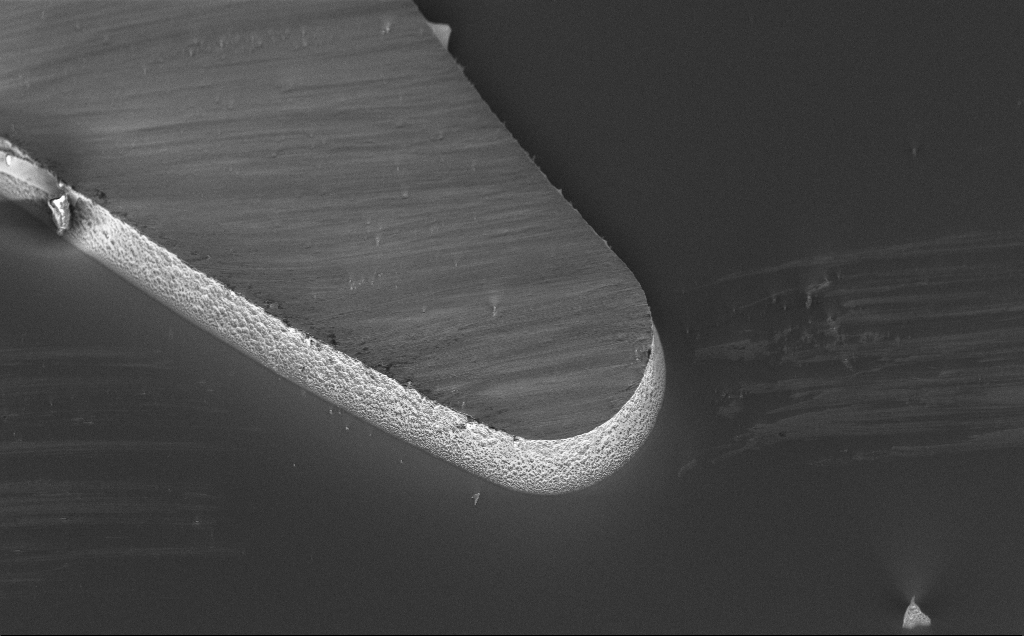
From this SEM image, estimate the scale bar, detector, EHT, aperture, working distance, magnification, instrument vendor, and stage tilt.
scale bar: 20000 nm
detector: InLens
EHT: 5 kV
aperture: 30 µm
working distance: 8 mm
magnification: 1.13 K X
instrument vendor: Zeiss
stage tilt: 45°